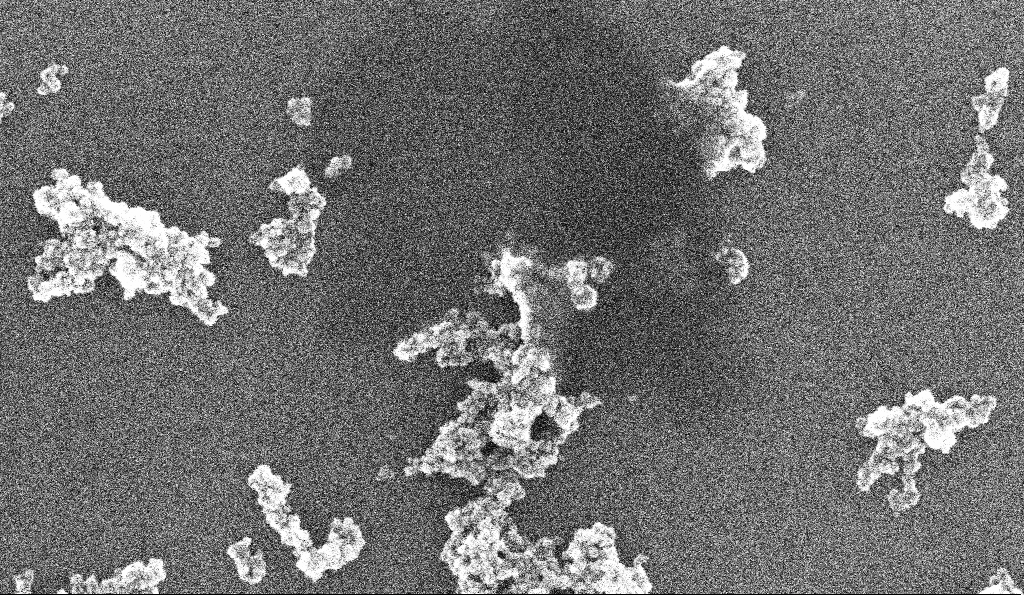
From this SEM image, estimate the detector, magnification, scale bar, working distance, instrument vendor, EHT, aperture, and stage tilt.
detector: InLens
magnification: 194.83 K X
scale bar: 100 nm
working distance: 5.2 mm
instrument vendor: Zeiss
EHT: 10 kV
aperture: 30 µm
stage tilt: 0°